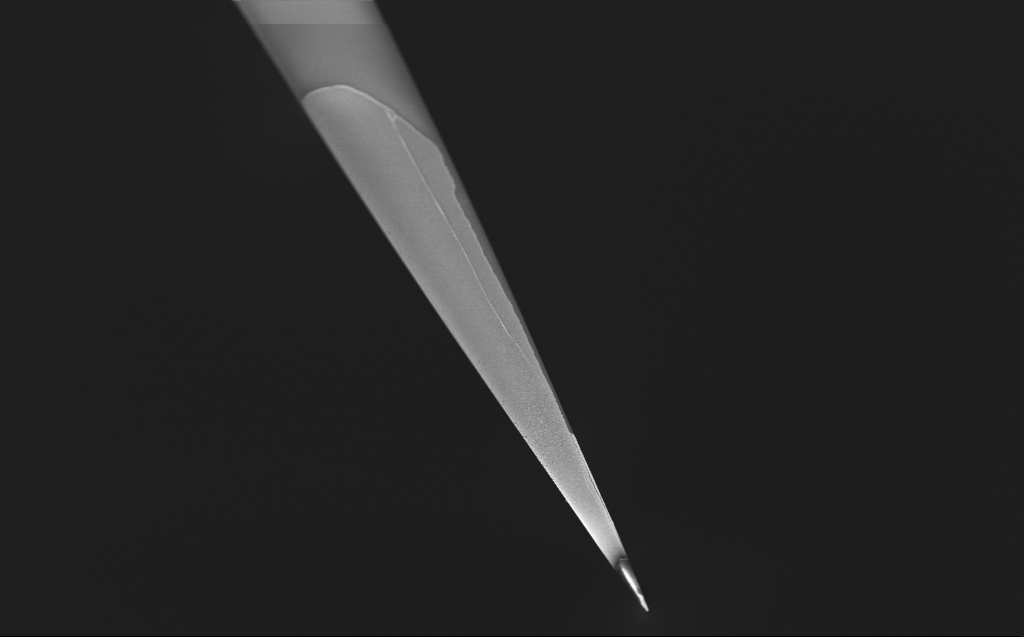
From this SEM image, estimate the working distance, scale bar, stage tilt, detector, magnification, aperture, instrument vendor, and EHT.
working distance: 4 mm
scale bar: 10000 nm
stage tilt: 45°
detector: InLens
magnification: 2.5 K X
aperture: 30 µm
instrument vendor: Zeiss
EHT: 0.8 kV